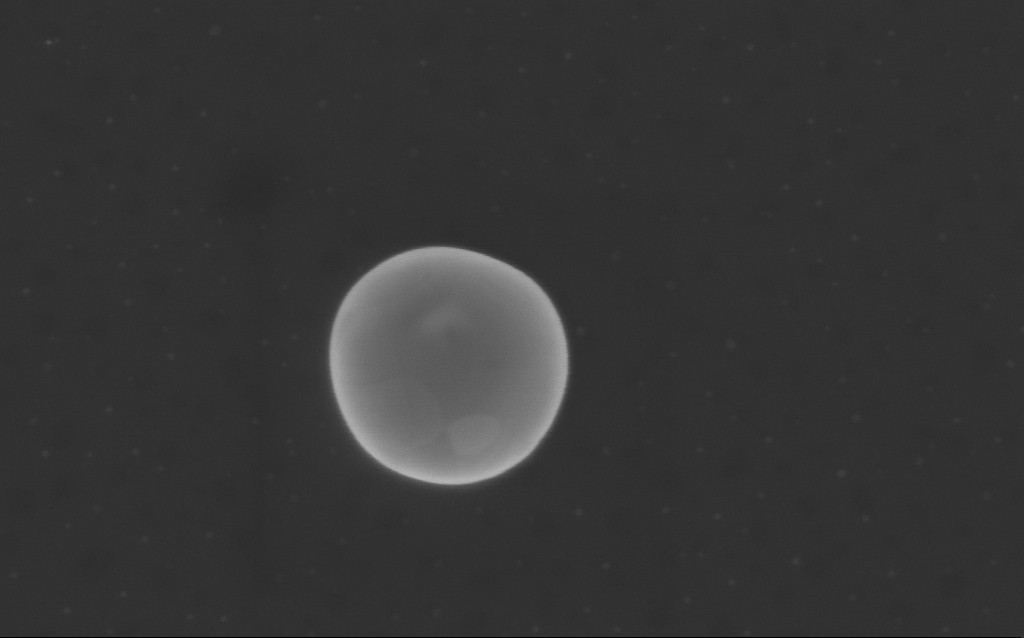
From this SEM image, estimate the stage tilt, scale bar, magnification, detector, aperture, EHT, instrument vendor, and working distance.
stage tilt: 0°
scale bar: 200 nm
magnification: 119.72 K X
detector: InLens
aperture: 30 µm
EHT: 3 kV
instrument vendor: Zeiss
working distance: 4 mm